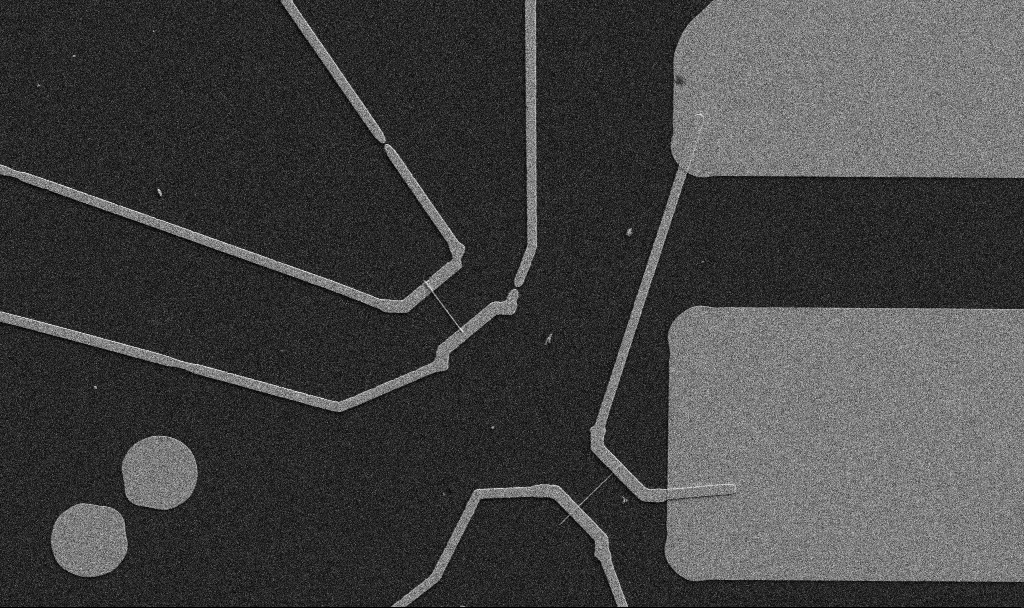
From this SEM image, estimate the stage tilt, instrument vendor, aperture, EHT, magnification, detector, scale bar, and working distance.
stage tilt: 0°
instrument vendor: Zeiss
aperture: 30 µm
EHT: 5 kV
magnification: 5 K X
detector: SE2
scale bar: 10000 nm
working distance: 10.7 mm